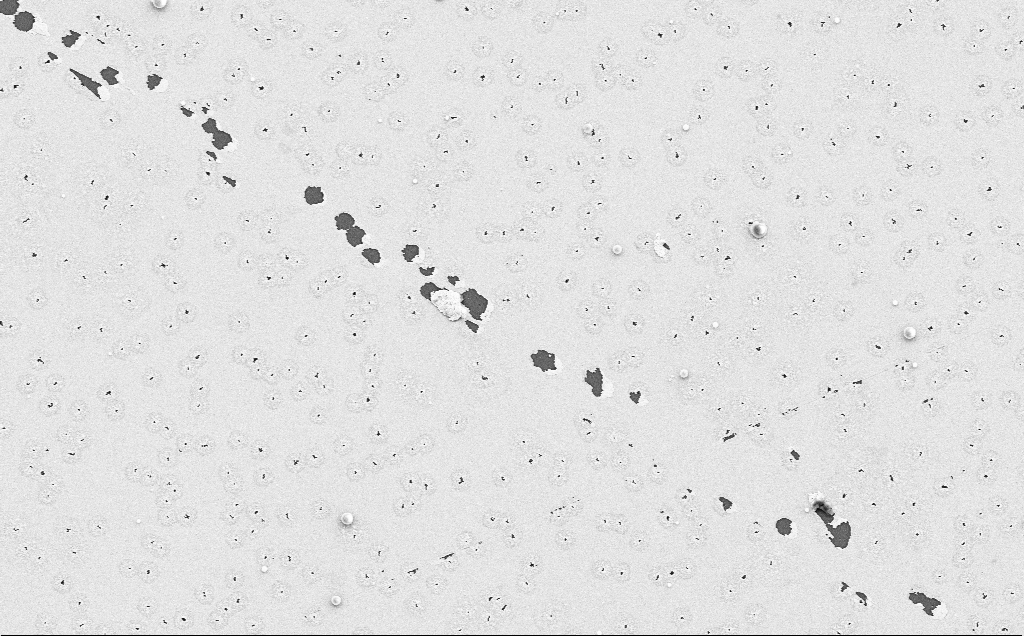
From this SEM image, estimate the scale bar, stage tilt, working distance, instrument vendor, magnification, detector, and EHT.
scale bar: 10000 nm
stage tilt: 0°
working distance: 12 mm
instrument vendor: Zeiss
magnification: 4.2 K X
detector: SE2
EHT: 5 kV